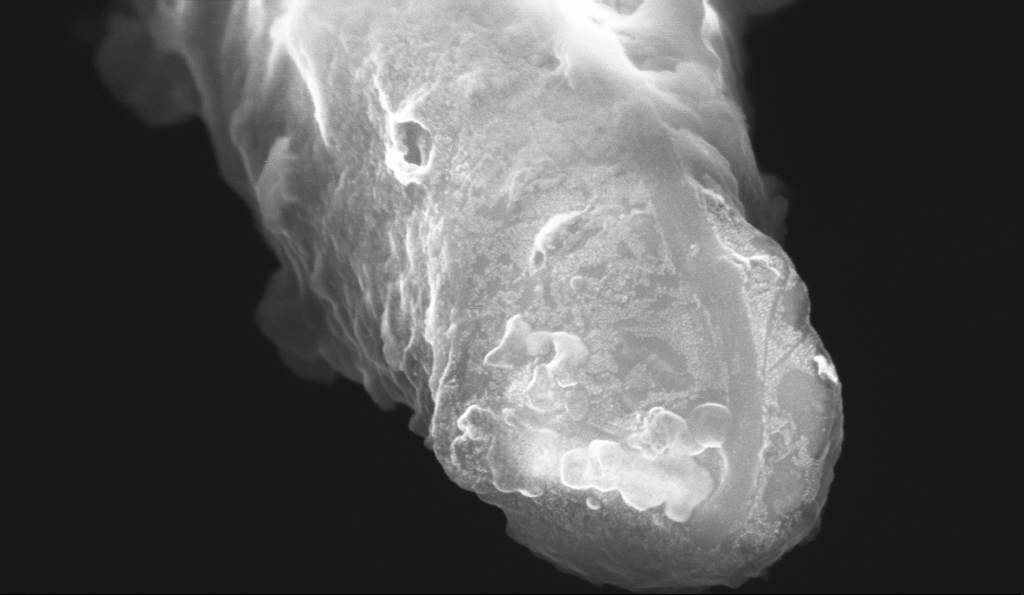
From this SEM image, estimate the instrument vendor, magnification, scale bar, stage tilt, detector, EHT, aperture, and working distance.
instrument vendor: Zeiss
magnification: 163.58 K X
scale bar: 100 nm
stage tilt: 70°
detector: InLens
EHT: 10 kV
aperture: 30 µm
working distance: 5.1 mm